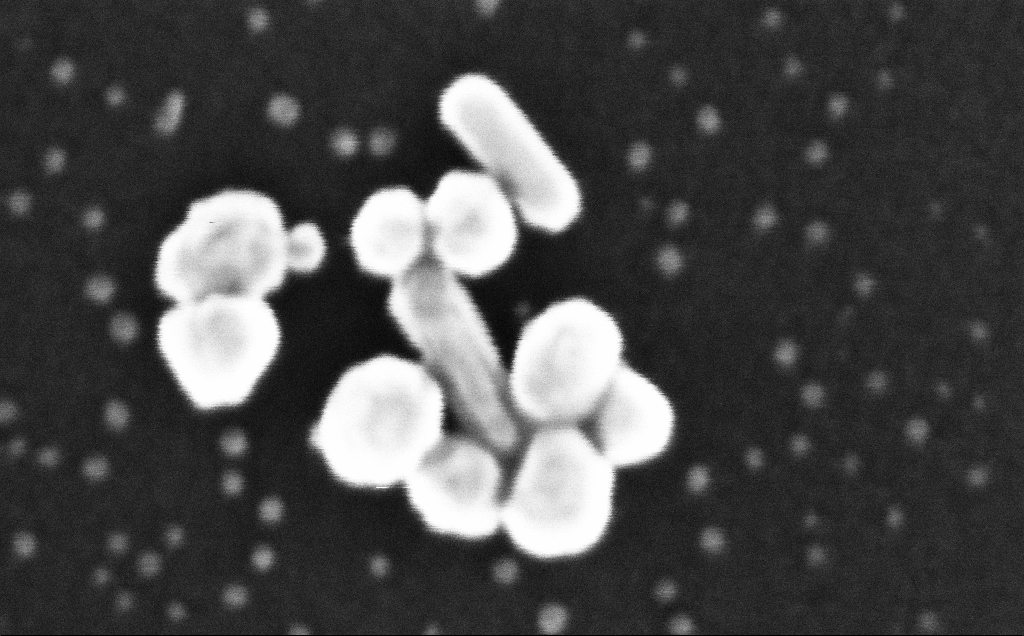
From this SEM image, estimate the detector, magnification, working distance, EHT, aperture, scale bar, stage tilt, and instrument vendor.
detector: InLens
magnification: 1098.36 K X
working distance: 2 mm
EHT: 20 kV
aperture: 30 µm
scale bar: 20 nm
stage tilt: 0°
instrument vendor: Zeiss